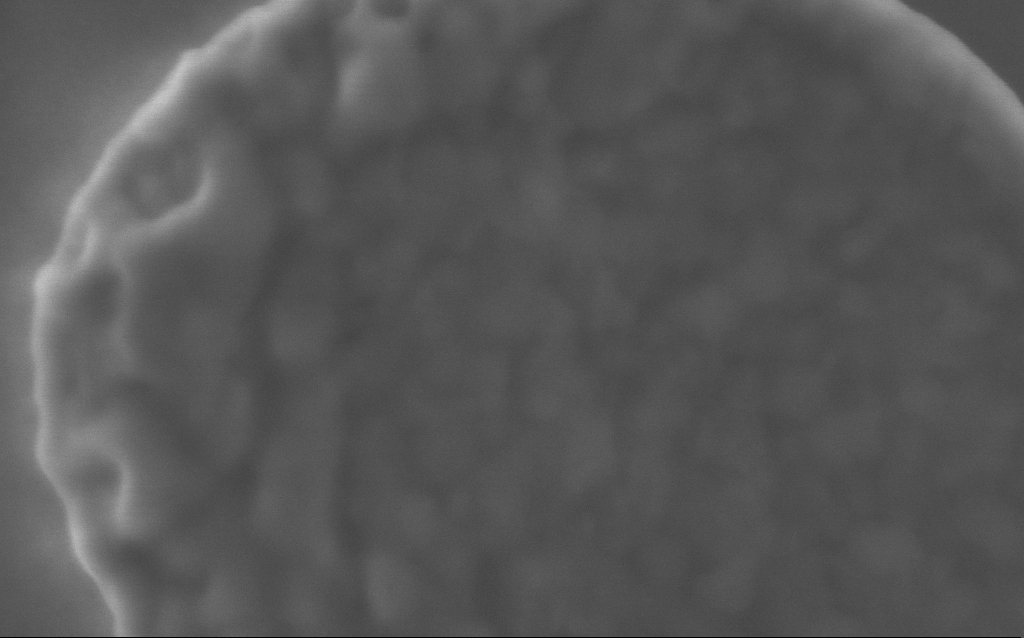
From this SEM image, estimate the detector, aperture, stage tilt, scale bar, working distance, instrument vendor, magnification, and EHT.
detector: InLens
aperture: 30 µm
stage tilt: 0°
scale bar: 100 nm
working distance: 3 mm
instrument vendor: Zeiss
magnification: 234.86 K X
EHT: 5 kV